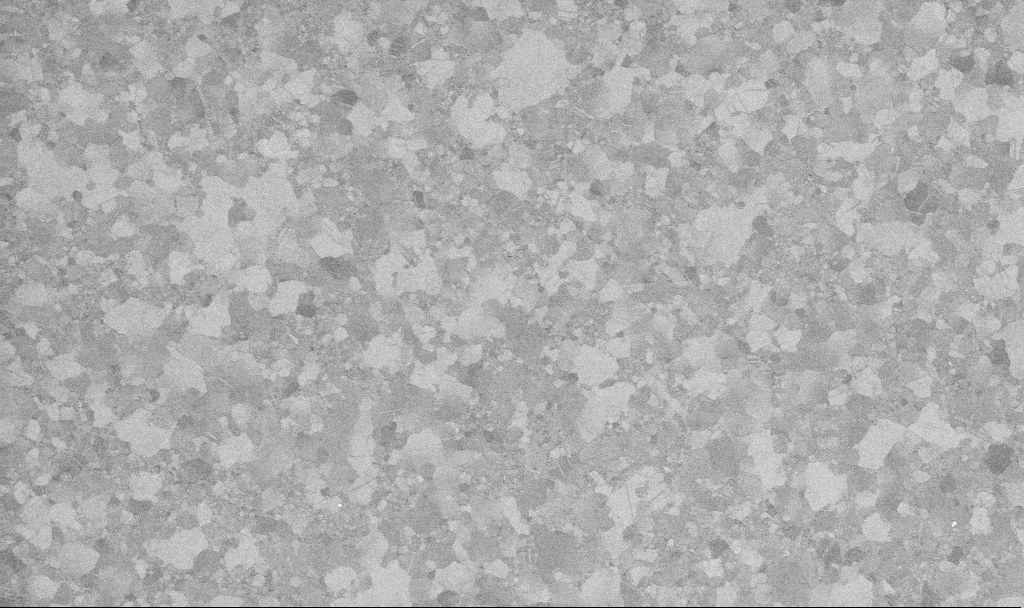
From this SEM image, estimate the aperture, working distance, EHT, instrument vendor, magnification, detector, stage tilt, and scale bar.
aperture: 30 µm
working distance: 3.4 mm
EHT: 10 kV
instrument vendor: Zeiss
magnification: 50 K X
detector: InLens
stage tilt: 0°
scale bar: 1000 nm